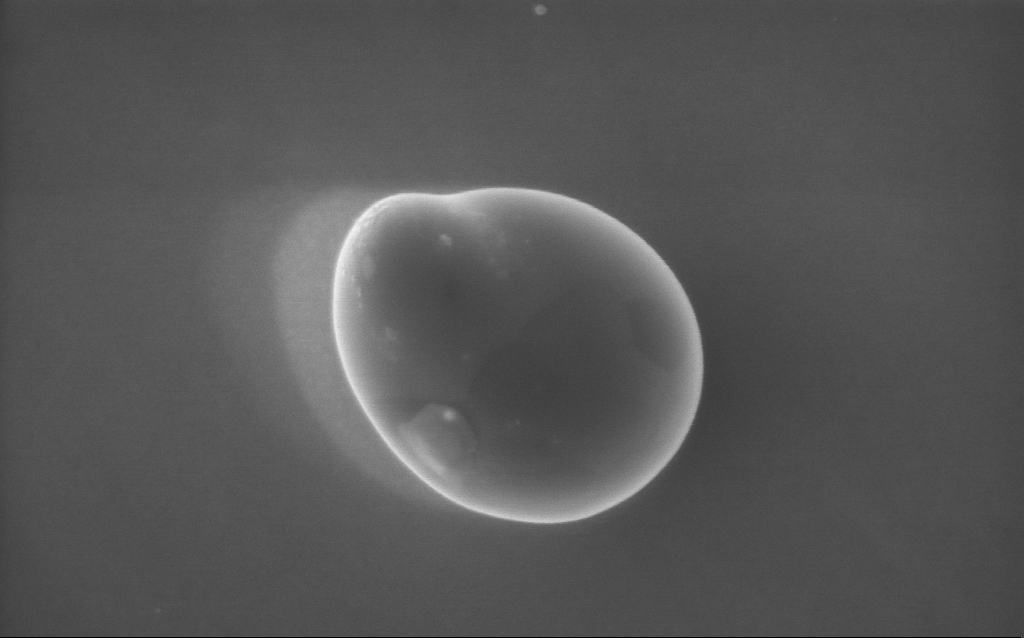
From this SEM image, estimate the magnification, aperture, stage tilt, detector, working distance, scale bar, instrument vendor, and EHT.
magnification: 80 K X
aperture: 30 µm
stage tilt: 0°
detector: InLens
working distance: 3 mm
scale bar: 200 nm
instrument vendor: Zeiss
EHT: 5 kV